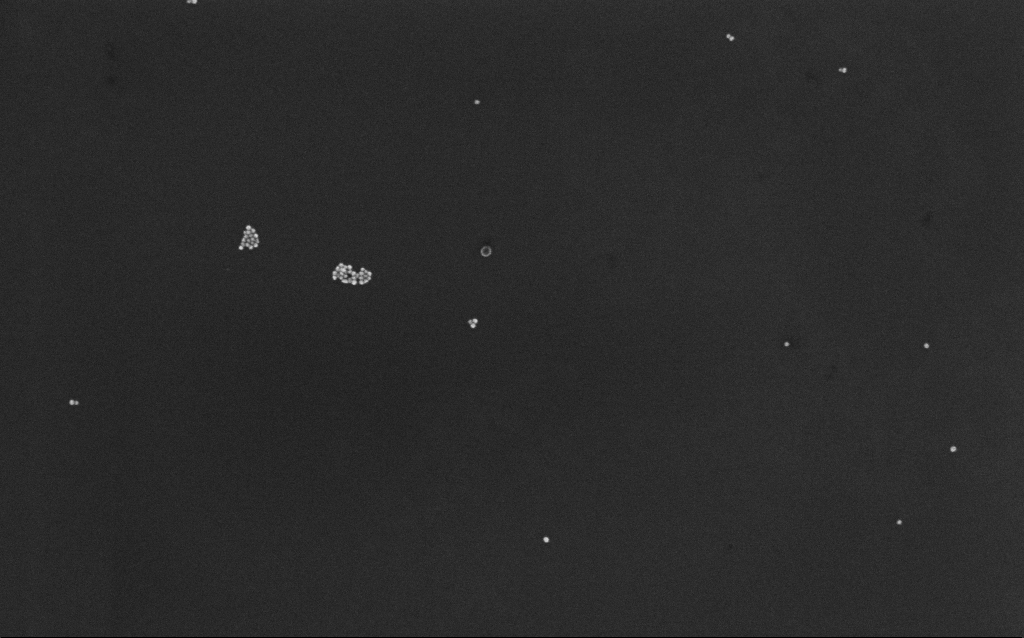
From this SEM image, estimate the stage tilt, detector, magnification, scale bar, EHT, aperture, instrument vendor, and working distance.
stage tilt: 0°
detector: InLens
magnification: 100 K X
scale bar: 200 nm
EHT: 10 kV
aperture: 30 µm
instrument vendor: Zeiss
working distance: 7 mm